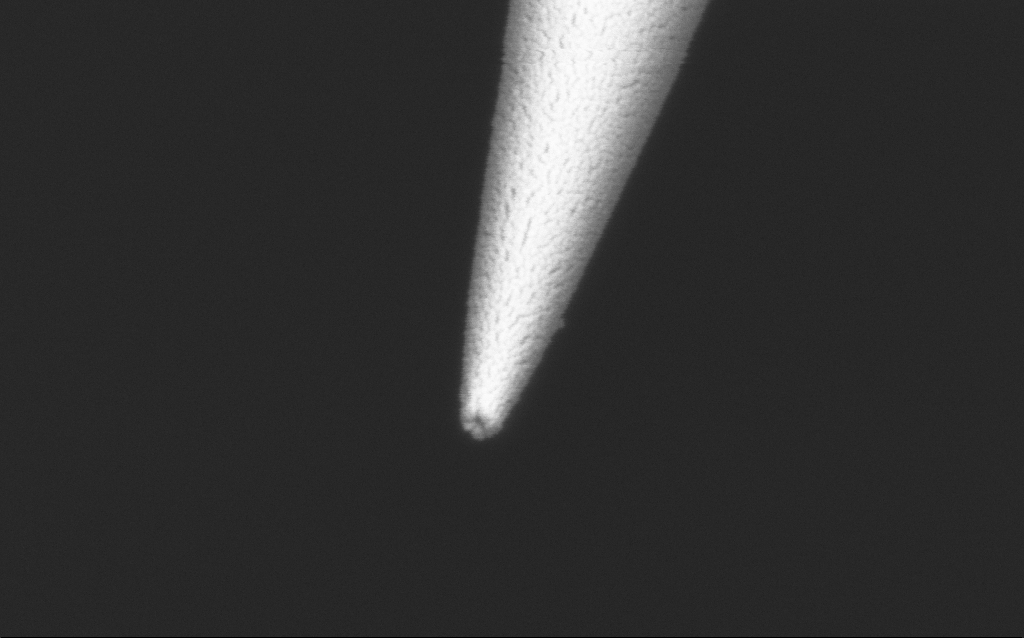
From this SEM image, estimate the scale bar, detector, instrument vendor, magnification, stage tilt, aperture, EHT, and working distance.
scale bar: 200 nm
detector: InLens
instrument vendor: Zeiss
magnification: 150 K X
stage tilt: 45°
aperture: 30 µm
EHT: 2 kV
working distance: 7.6 mm